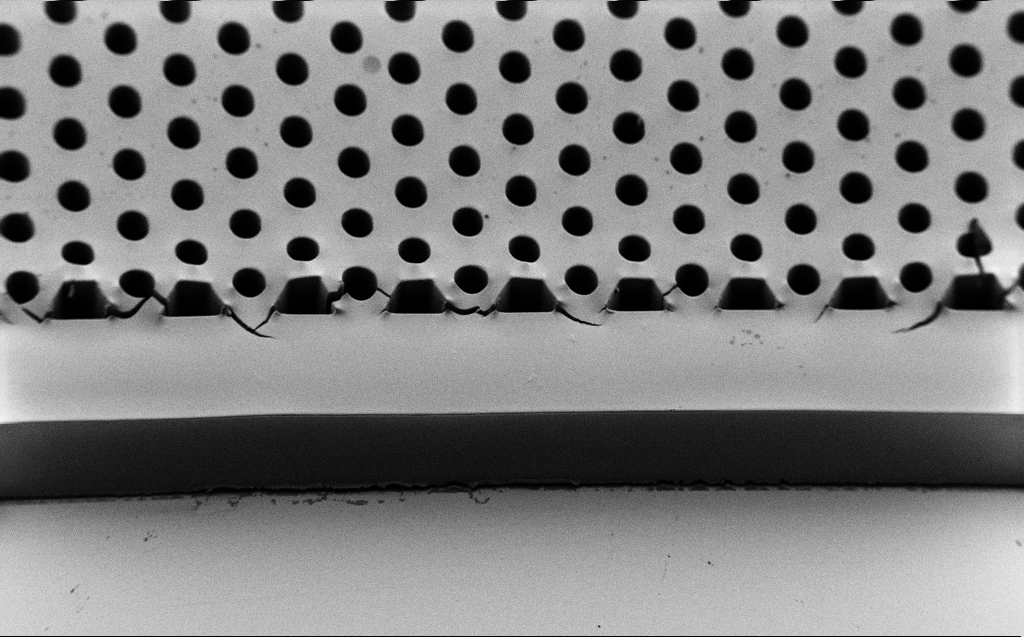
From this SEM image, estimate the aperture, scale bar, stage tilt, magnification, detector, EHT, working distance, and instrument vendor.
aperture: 30 µm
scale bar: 100000 nm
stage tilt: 45°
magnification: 0.335 K X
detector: SE2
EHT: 1.7 kV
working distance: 8 mm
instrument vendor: Zeiss